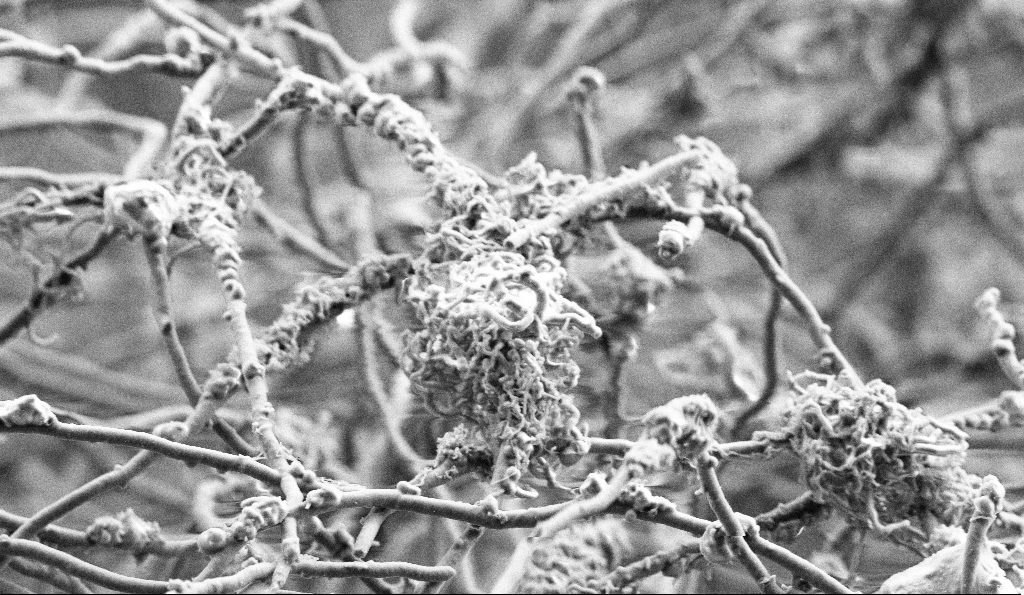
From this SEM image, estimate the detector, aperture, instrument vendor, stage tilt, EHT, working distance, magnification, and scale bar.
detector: SE2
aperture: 30 µm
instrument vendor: Zeiss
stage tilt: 0°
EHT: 3 kV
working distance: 5 mm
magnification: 8 K X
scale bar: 2000 nm